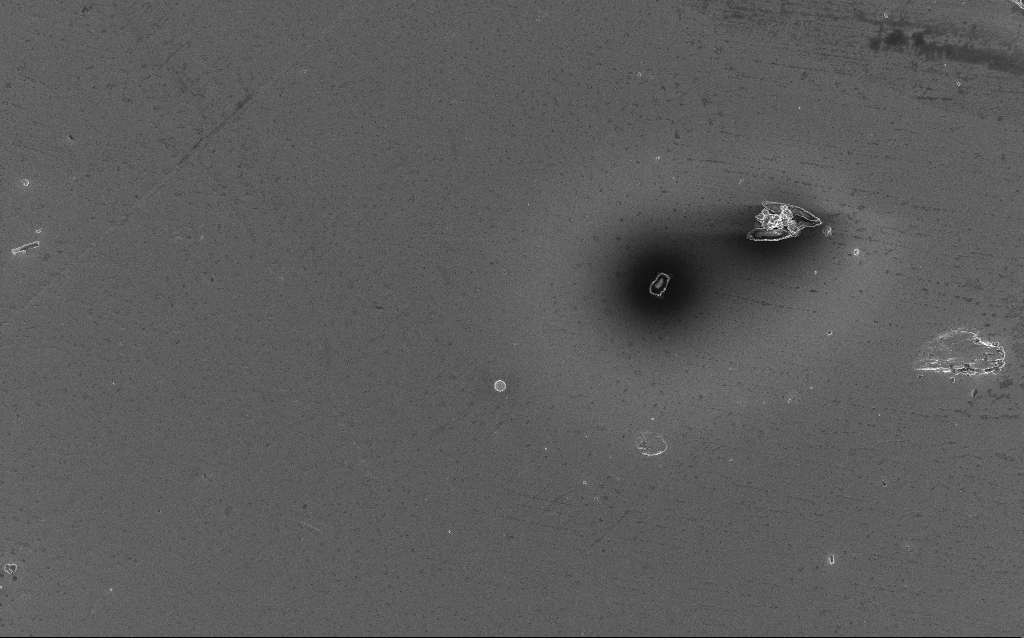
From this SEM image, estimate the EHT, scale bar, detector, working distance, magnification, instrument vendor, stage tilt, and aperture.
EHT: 5 kV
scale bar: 20000 nm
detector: InLens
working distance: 5 mm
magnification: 1.1 K X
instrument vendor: Zeiss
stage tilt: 0°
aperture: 30 µm